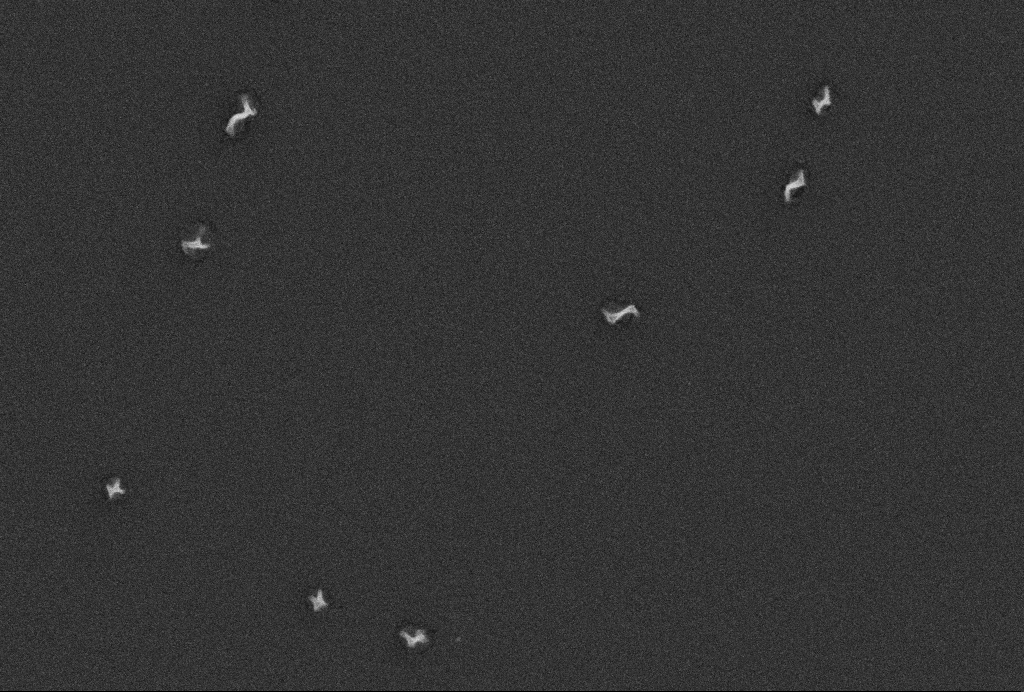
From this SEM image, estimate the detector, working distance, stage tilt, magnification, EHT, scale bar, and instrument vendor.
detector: SE2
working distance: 2.6 mm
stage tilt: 45°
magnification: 100 K X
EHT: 5 kV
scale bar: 200 nm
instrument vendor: Zeiss